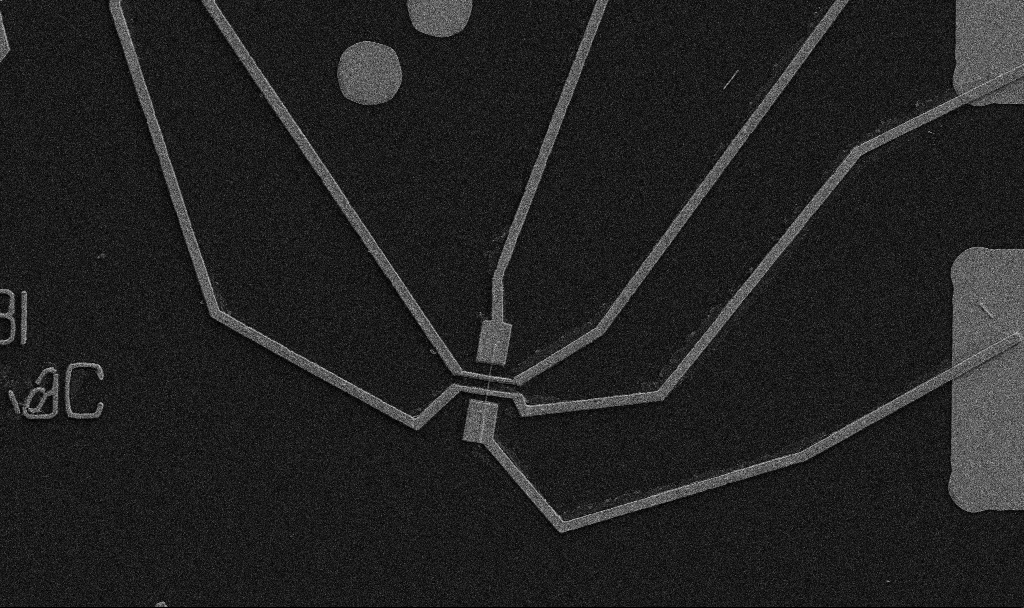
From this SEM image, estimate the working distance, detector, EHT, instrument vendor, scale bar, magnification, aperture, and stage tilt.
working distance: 10.7 mm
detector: SE2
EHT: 5 kV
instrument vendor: Zeiss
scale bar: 10000 nm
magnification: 5 K X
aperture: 30 µm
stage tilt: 0°